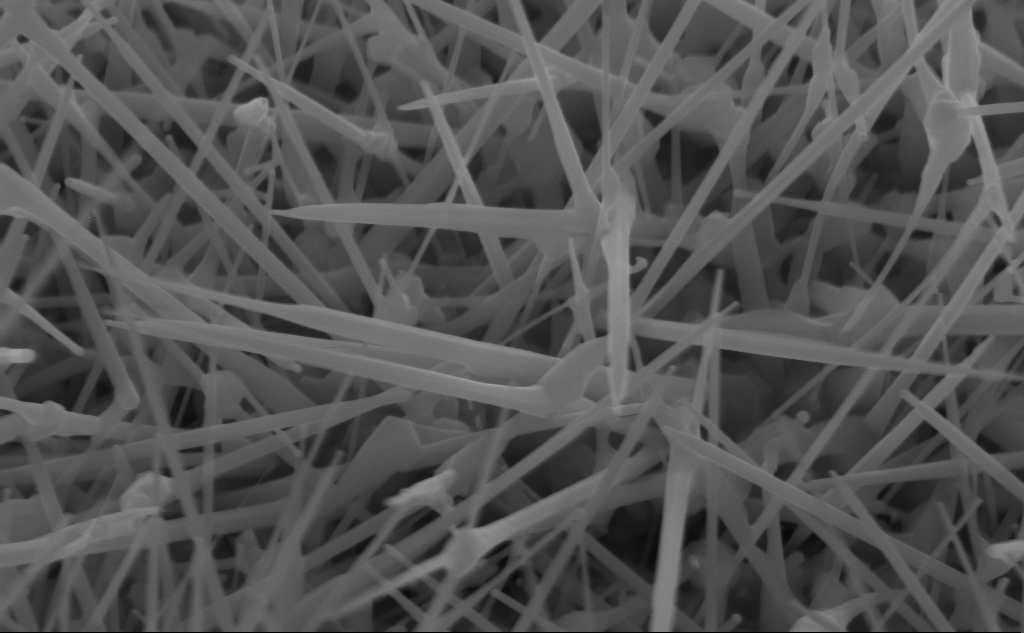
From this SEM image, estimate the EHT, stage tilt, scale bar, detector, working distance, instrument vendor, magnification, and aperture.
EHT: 10 kV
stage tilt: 45°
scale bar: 200 nm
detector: InLens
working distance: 4 mm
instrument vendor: Zeiss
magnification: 80 K X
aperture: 30 µm